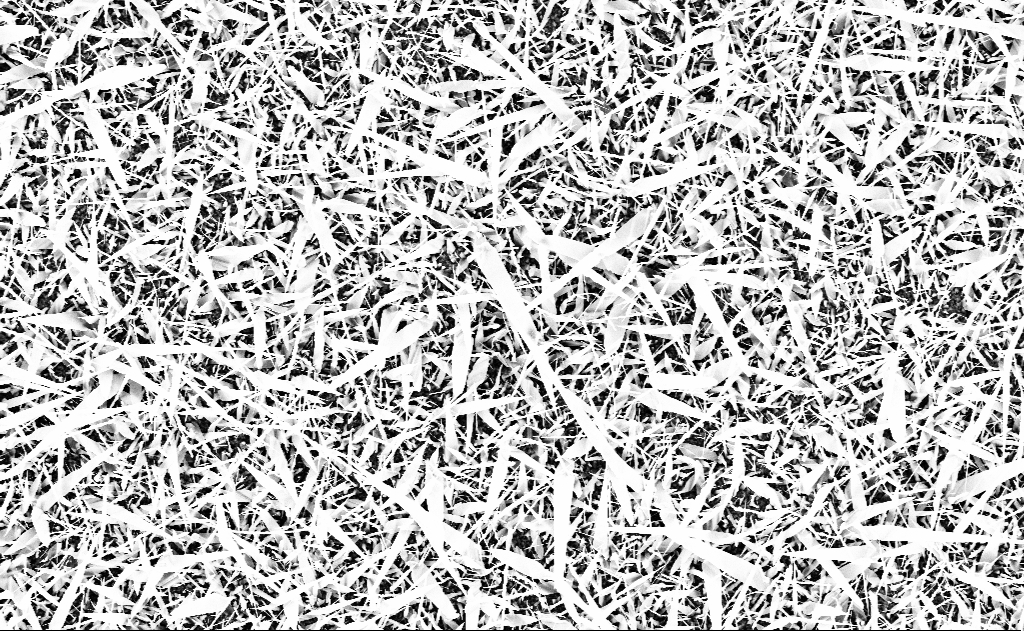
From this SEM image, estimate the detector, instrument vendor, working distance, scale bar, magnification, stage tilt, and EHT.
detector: InLens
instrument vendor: Zeiss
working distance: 13 mm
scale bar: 10000 nm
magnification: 5 K X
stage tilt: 0°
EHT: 10 kV